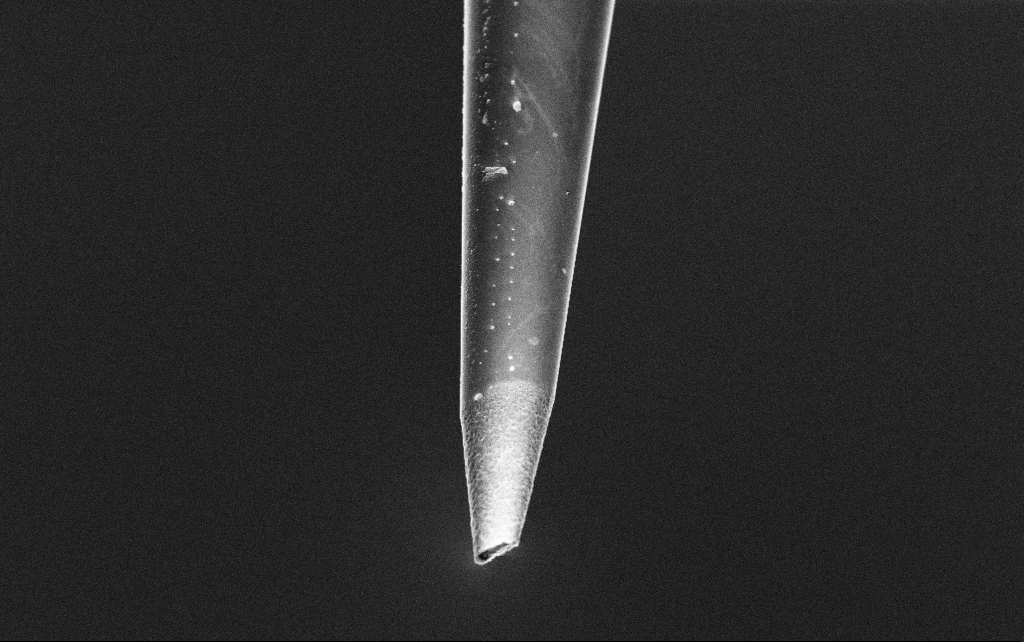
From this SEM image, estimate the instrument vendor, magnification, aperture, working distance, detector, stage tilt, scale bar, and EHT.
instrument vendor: Zeiss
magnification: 15 K X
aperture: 30 µm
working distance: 7.7 mm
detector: InLens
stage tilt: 45°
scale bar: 2000 nm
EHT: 3 kV